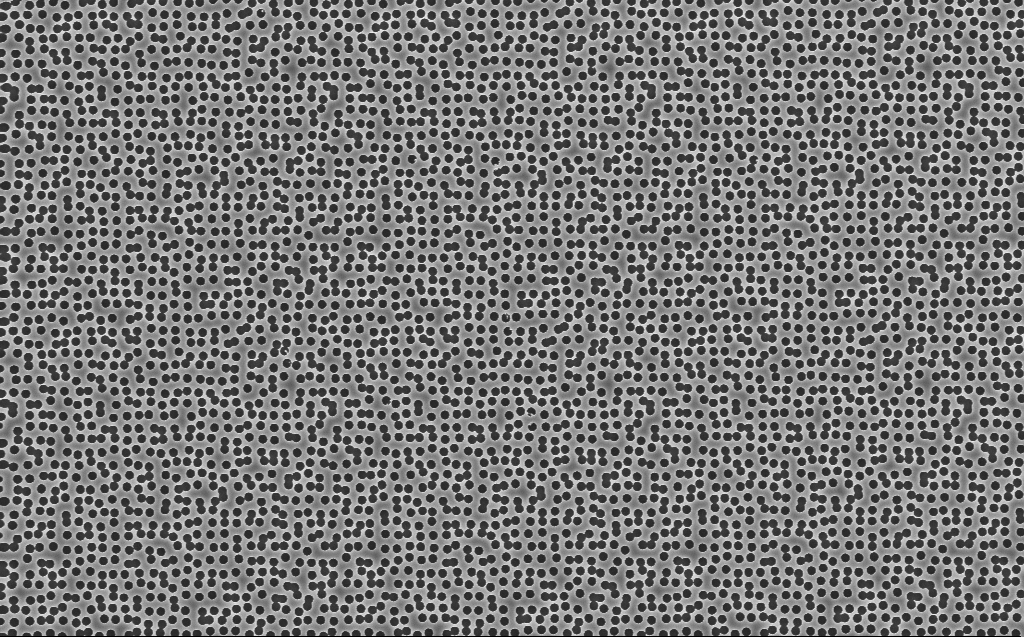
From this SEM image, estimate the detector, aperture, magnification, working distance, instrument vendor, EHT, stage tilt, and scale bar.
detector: InLens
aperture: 30 µm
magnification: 11.22 K X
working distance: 7 mm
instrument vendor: Zeiss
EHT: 5 kV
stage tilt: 0°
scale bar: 2000 nm